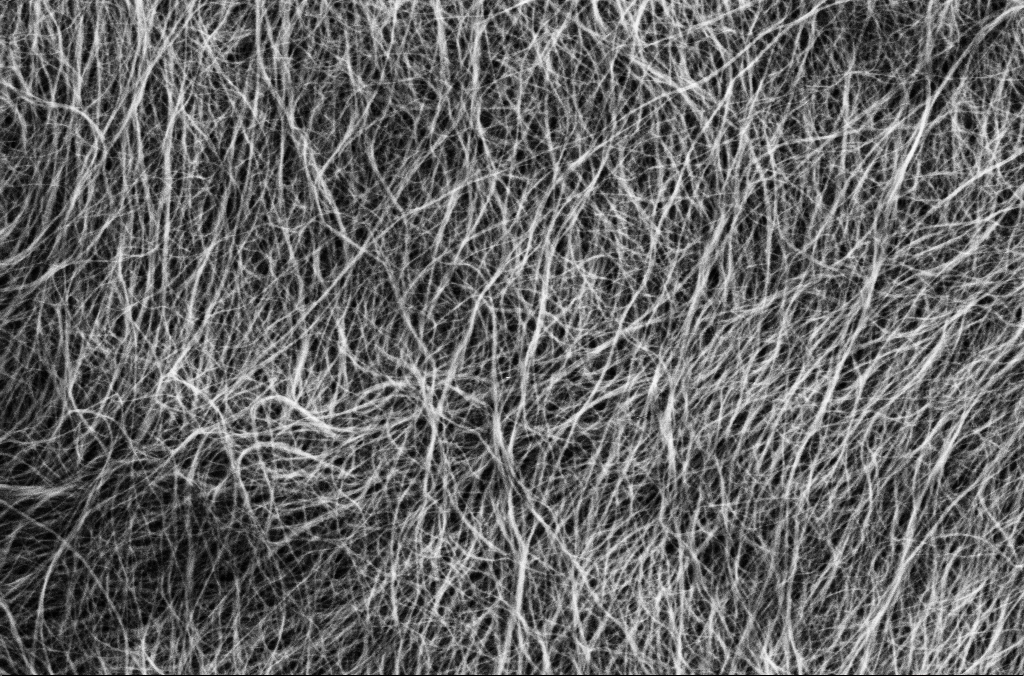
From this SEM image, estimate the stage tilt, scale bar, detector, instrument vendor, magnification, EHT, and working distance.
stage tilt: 0°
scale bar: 1000 nm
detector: SE2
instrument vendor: Zeiss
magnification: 50 K X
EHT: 1.8 kV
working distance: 5.7 mm